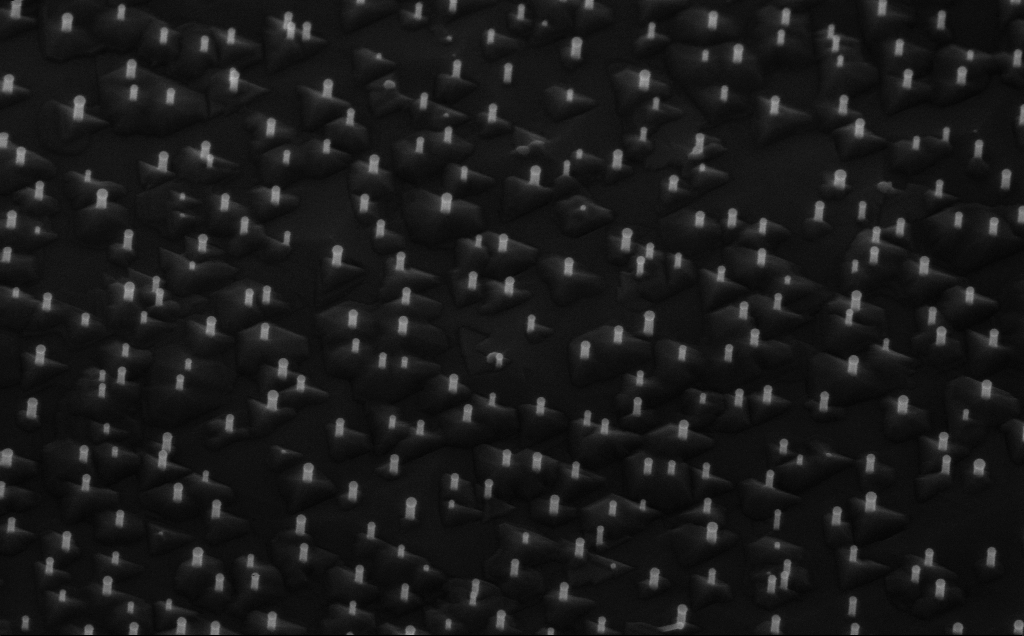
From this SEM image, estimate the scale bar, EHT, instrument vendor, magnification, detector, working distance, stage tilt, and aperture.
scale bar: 200 nm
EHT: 10 kV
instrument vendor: Zeiss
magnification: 80 K X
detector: InLens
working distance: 6 mm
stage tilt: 45°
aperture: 30 µm